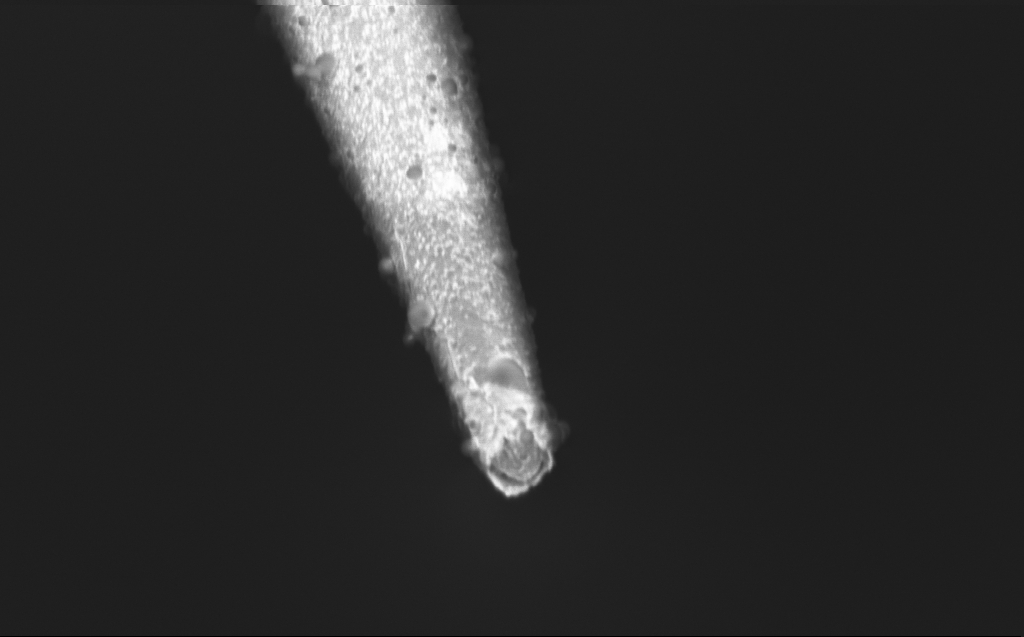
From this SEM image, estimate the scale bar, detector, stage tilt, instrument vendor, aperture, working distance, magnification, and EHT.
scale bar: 1000 nm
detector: InLens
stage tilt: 45°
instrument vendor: Zeiss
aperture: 30 µm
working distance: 4 mm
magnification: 50 K X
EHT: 0.8 kV